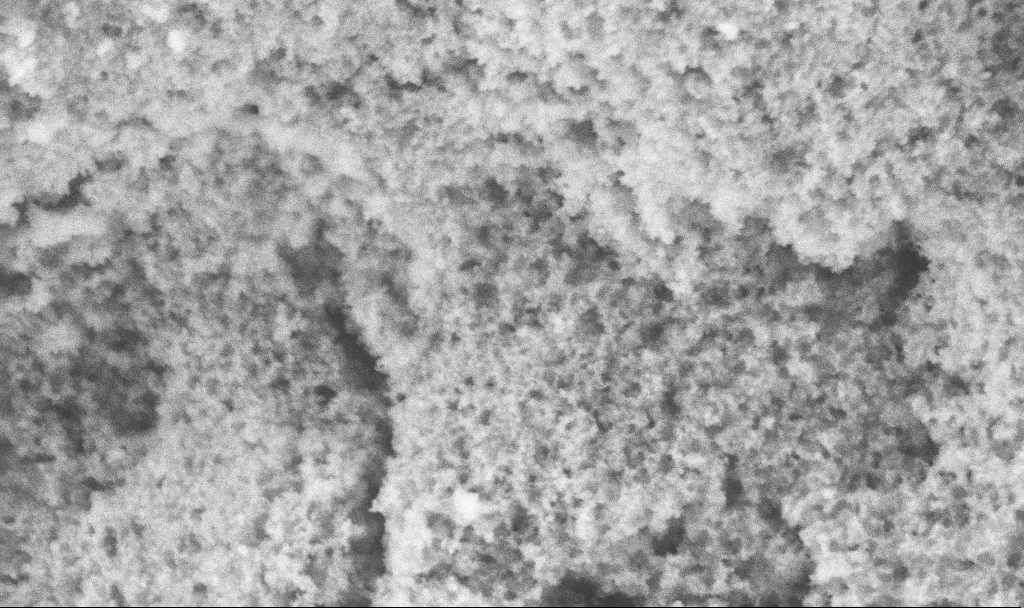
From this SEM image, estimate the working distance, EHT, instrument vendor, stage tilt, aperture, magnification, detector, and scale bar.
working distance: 2.7 mm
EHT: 5 kV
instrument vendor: Zeiss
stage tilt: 0°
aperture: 30 µm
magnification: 53.67 K X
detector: SE2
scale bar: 1000 nm